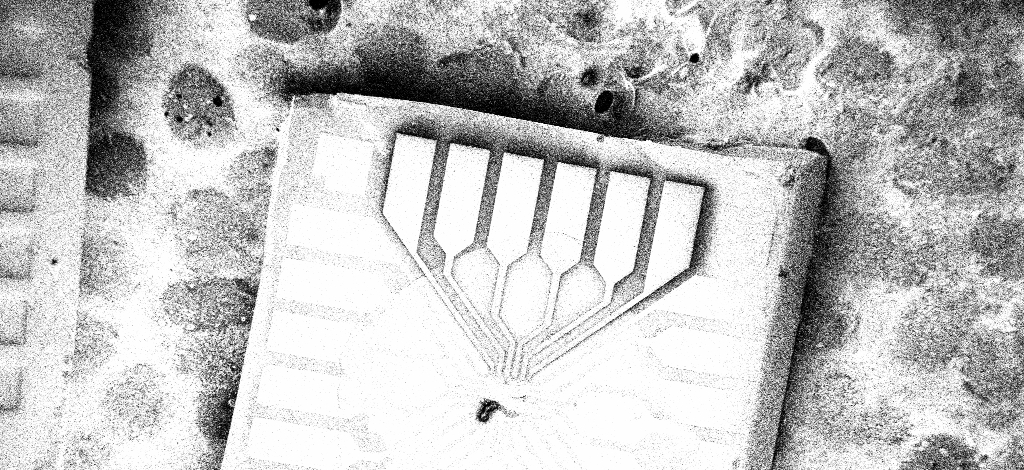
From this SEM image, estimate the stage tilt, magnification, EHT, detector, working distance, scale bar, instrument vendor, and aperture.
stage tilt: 0°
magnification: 0.1 K X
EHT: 5 kV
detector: InLens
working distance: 8.6 mm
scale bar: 200000 nm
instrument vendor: Zeiss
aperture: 30 µm